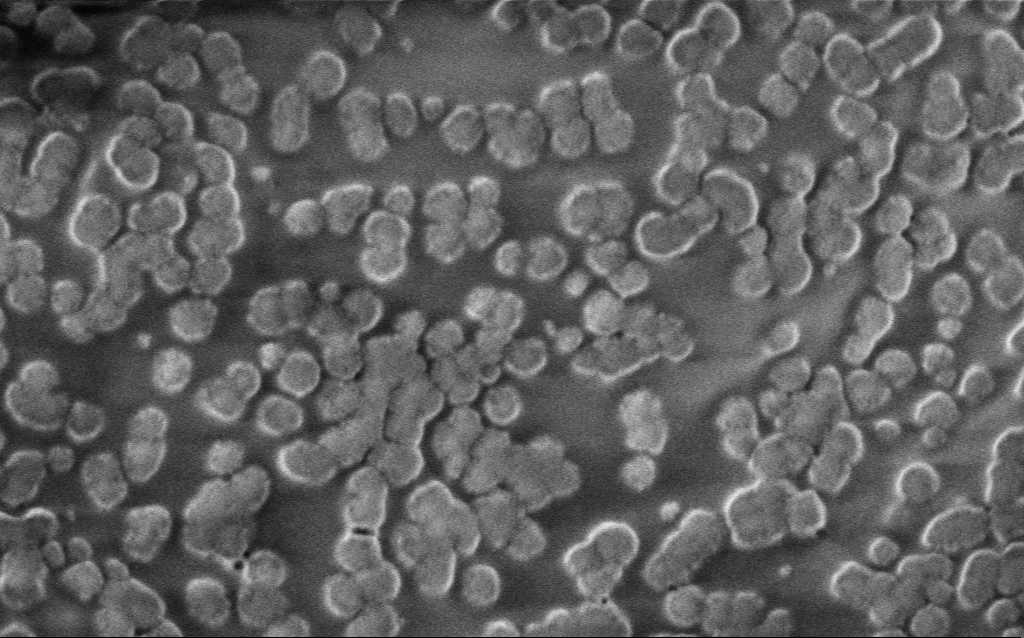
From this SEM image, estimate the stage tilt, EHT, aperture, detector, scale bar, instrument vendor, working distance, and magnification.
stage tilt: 0°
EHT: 1 kV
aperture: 30 µm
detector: InLens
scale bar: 200 nm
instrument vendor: Zeiss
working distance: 4 mm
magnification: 87.79 K X